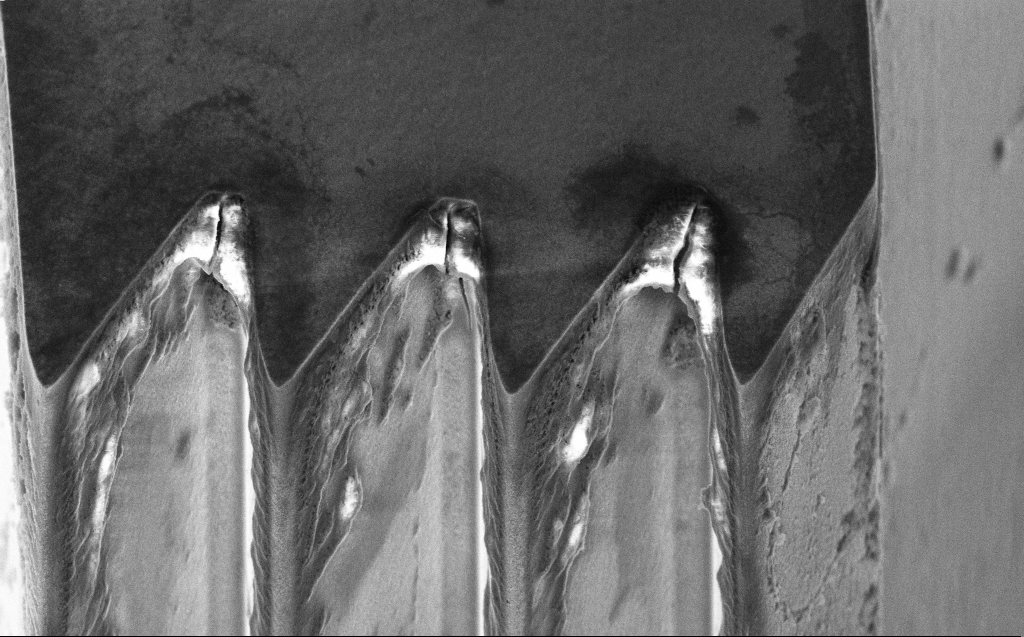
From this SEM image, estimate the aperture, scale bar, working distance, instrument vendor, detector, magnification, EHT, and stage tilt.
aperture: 30 µm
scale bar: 2000 nm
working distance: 7 mm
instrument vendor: Zeiss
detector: InLens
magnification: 7.55 K X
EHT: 5 kV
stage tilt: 45°